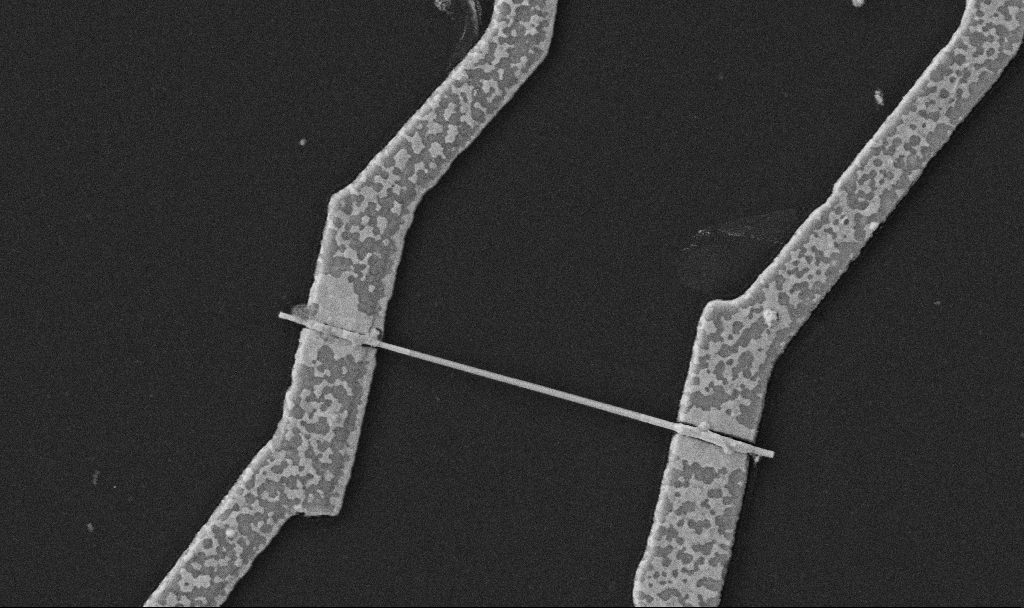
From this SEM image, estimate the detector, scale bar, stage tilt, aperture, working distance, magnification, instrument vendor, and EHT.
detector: SE2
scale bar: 1000 nm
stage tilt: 0°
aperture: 30 µm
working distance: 10.7 mm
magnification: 30 K X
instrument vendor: Zeiss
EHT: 5 kV